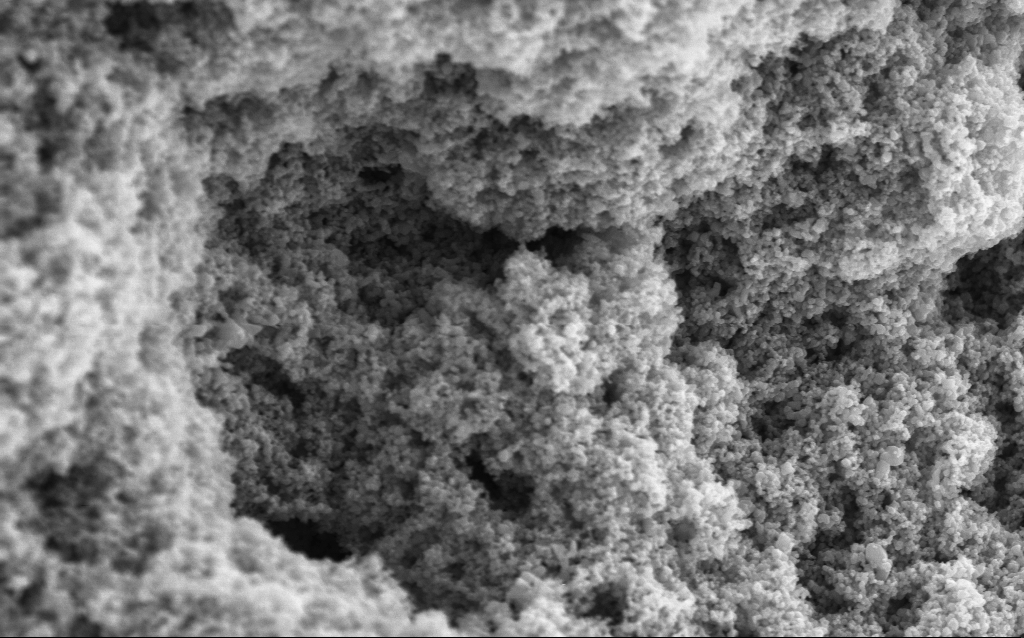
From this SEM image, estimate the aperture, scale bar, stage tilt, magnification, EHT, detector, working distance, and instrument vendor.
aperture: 30 µm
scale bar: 1000 nm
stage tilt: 0°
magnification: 68.65 K X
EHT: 5 kV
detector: InLens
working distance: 4.5 mm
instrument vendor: Zeiss